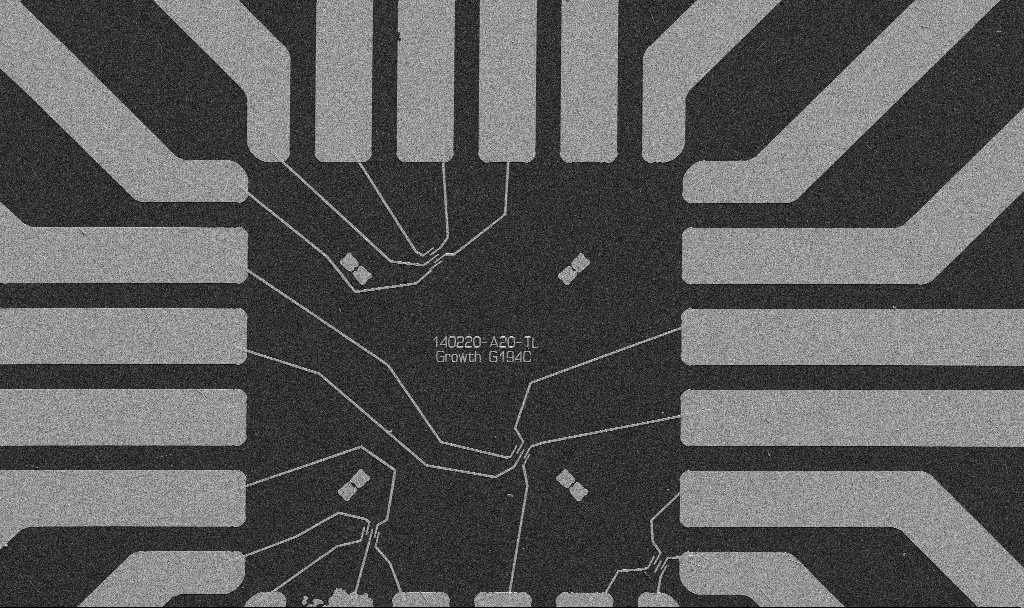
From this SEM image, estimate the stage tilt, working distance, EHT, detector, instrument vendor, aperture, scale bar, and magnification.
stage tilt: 0°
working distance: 10.7 mm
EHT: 5 kV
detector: SE2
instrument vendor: Zeiss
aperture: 30 µm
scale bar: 20000 nm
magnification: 1 K X